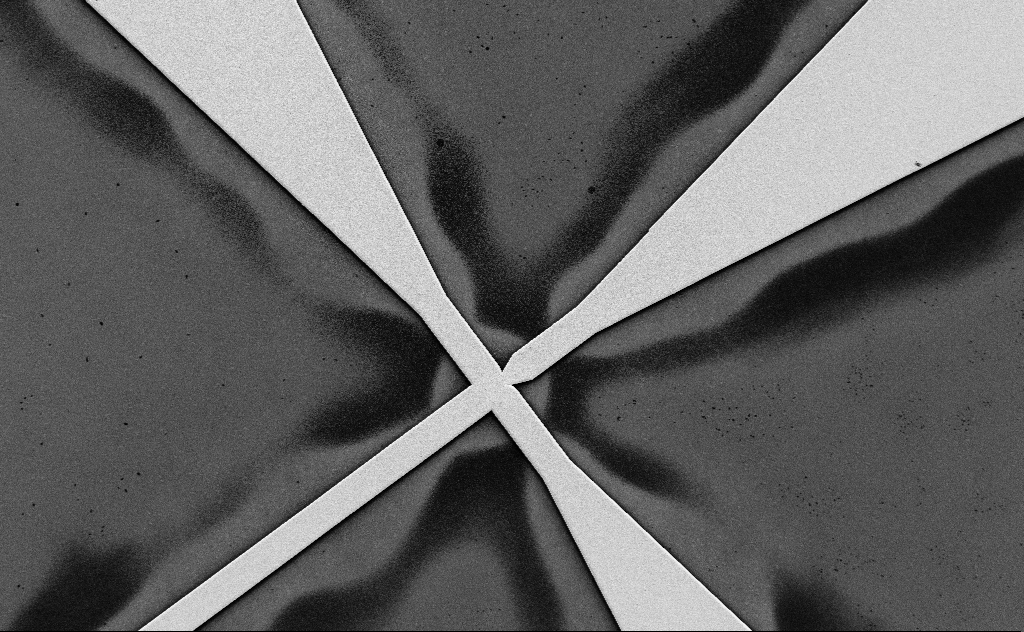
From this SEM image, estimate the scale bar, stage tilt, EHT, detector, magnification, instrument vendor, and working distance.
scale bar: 20000 nm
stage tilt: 0°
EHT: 3 kV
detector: SE2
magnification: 0.968 K X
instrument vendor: Zeiss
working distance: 13 mm